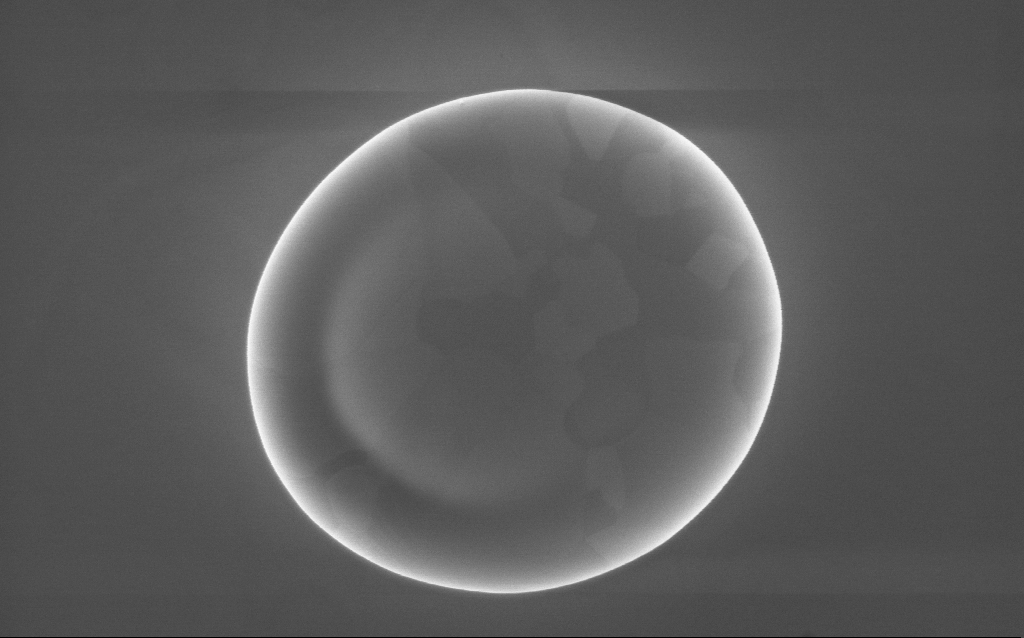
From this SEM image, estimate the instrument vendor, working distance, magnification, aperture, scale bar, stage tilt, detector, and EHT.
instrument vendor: Zeiss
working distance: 2 mm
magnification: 58 K X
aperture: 30 µm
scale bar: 1000 nm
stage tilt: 0°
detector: InLens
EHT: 10 kV